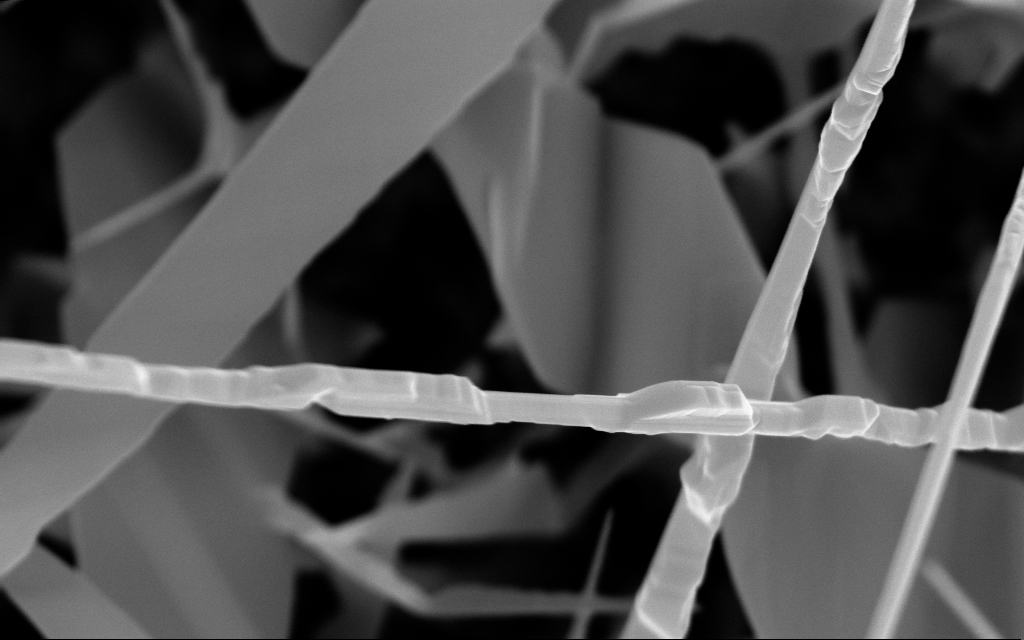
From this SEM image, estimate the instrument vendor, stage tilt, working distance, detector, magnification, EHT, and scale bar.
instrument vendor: Zeiss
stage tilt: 0°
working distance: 6 mm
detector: InLens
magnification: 94.35 K X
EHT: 10 kV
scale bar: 200 nm